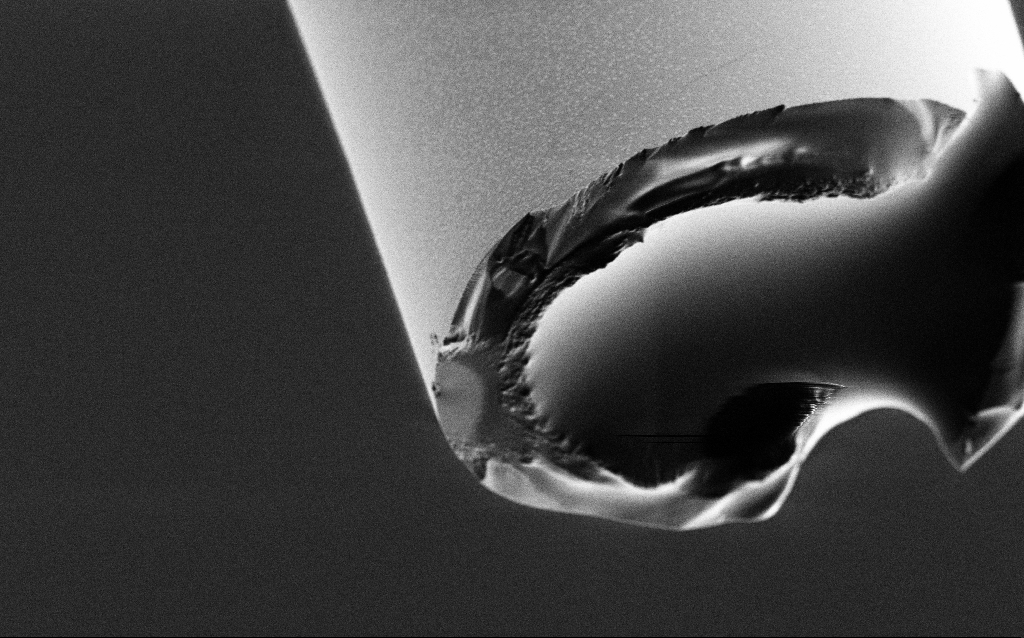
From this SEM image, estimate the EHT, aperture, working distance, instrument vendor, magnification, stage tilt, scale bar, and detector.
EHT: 1 kV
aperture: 30 µm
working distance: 6.6 mm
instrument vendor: Zeiss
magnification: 10 K X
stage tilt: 45°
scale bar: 2000 nm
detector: SE2